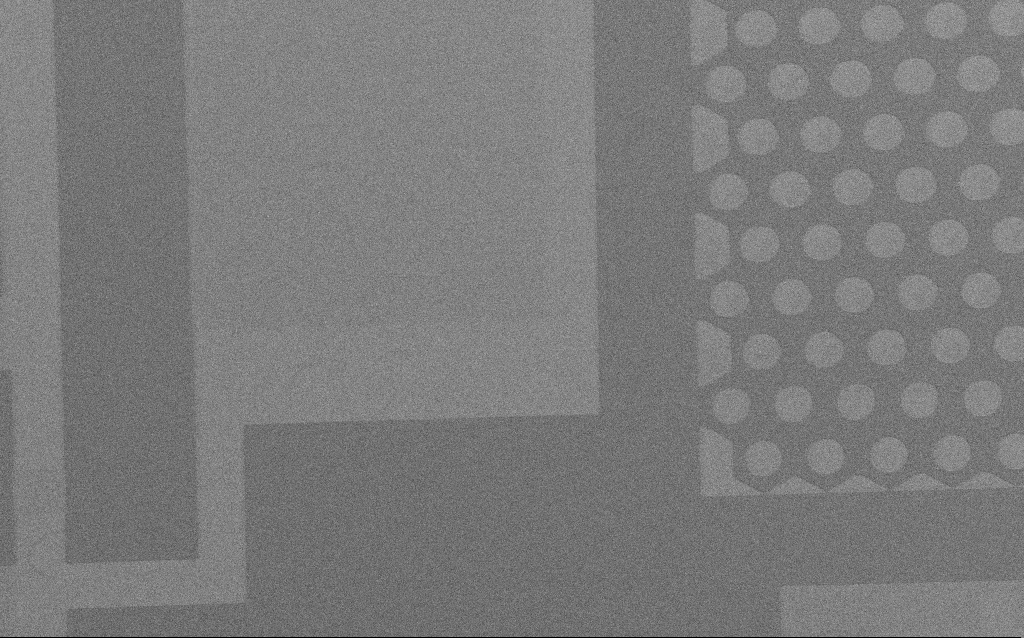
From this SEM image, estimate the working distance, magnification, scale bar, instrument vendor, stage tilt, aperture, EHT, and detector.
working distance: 4 mm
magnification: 0.331 K X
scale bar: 100000 nm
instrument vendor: Zeiss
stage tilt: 0°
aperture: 30 µm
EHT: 2 kV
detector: SE2